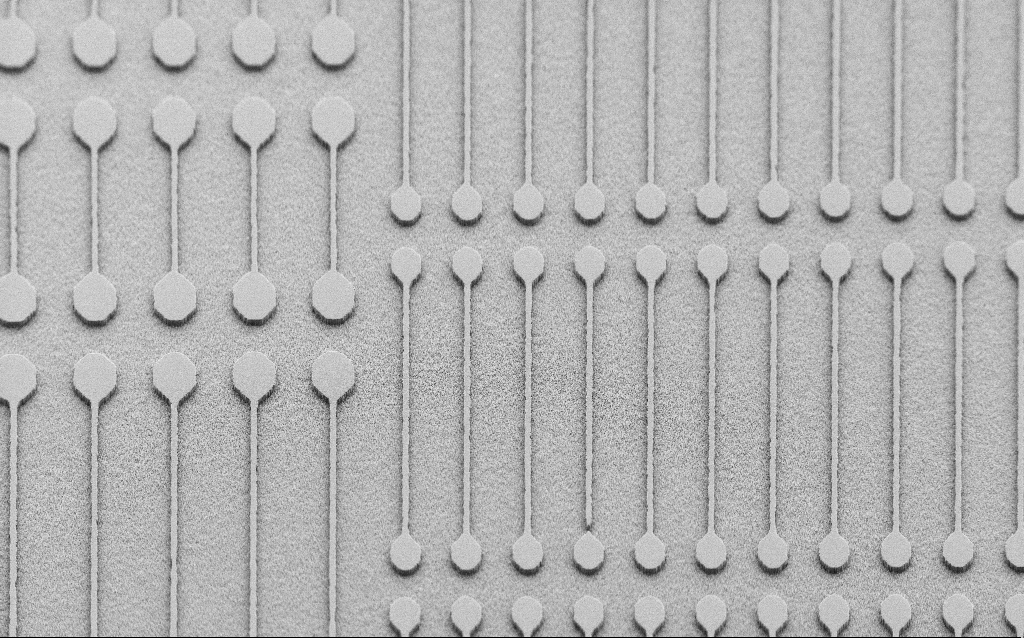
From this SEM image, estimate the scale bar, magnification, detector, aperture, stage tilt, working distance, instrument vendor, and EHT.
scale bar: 100000 nm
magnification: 0.47 K X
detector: SE2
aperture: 30 µm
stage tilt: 45°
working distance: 7 mm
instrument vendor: Zeiss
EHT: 2 kV